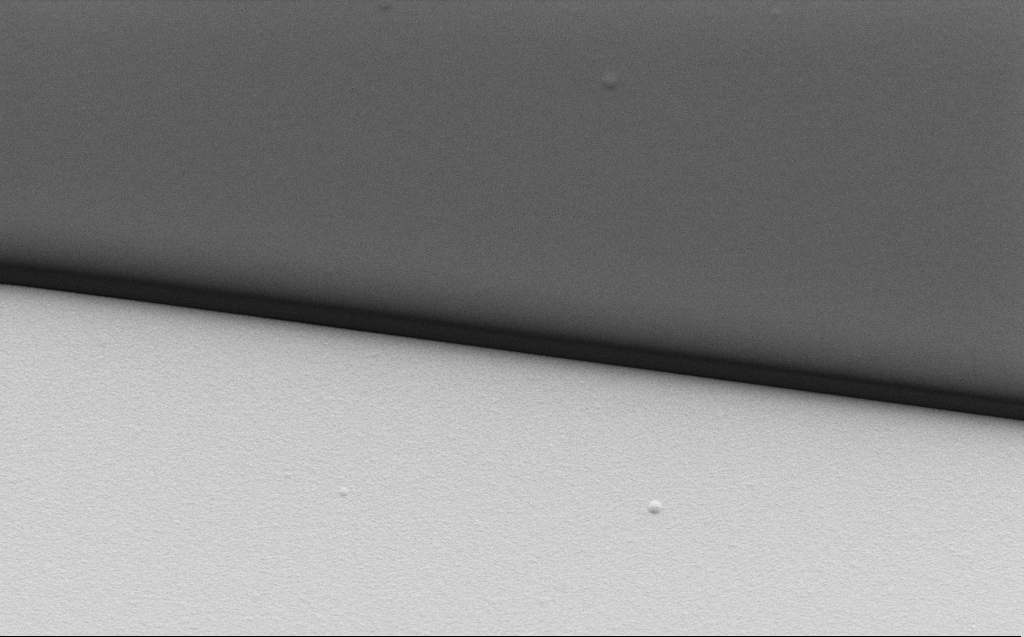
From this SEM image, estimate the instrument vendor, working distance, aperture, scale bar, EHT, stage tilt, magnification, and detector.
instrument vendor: Zeiss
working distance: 6 mm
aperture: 30 µm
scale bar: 10000 nm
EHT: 1.1 kV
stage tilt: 30°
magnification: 5.87 K X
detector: SE2